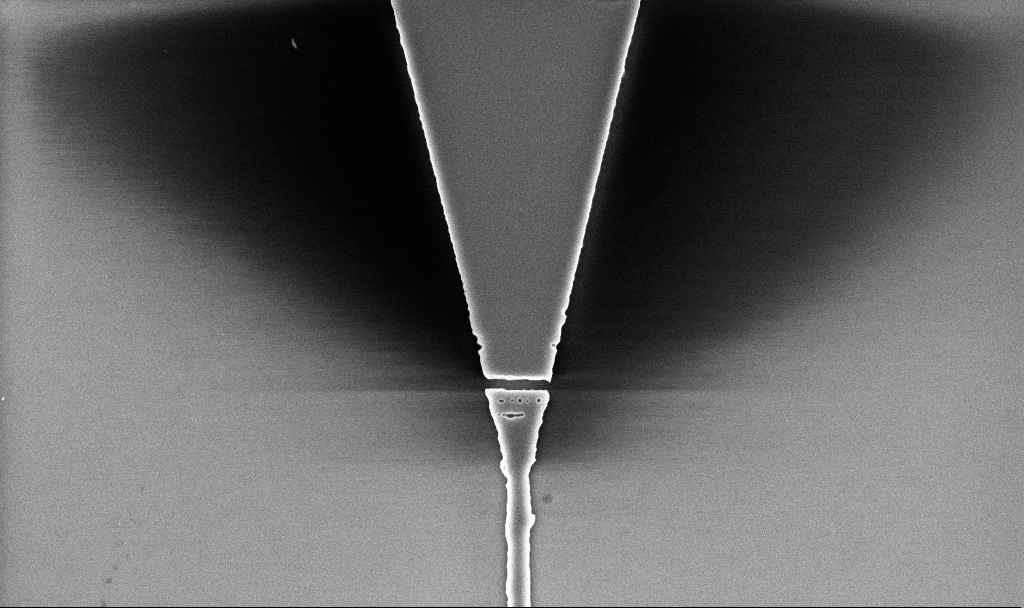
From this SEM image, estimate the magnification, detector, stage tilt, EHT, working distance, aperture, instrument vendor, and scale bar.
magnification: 16.61 K X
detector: InLens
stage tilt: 0°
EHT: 5 kV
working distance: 5.2 mm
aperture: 30 µm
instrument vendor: Zeiss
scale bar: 2000 nm